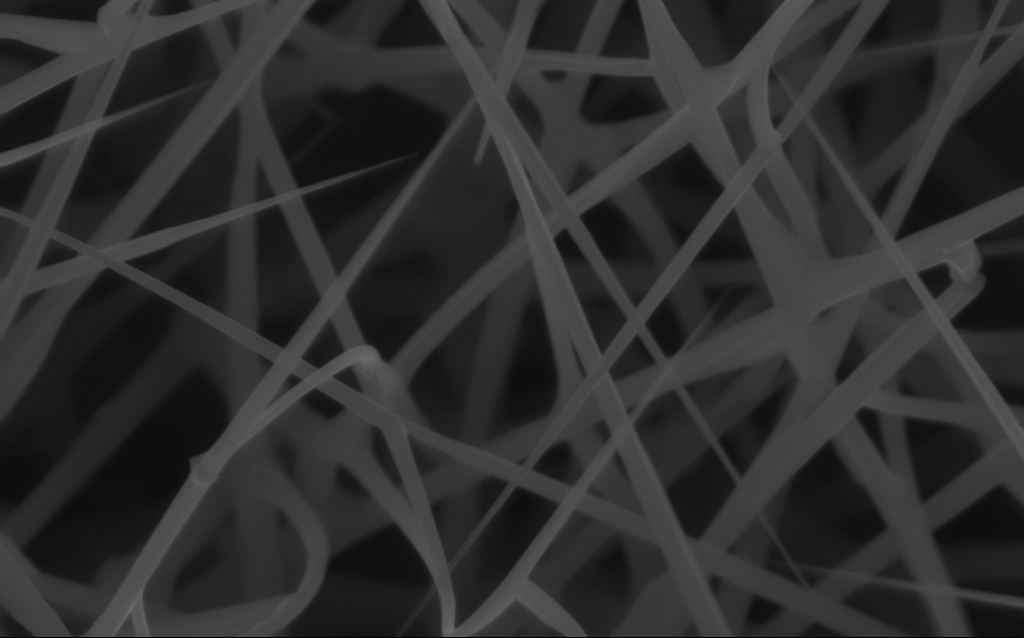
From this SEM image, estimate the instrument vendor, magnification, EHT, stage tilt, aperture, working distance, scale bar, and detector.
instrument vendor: Zeiss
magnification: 80 K X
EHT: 10 kV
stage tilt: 0°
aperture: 30 µm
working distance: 6 mm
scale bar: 200 nm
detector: InLens